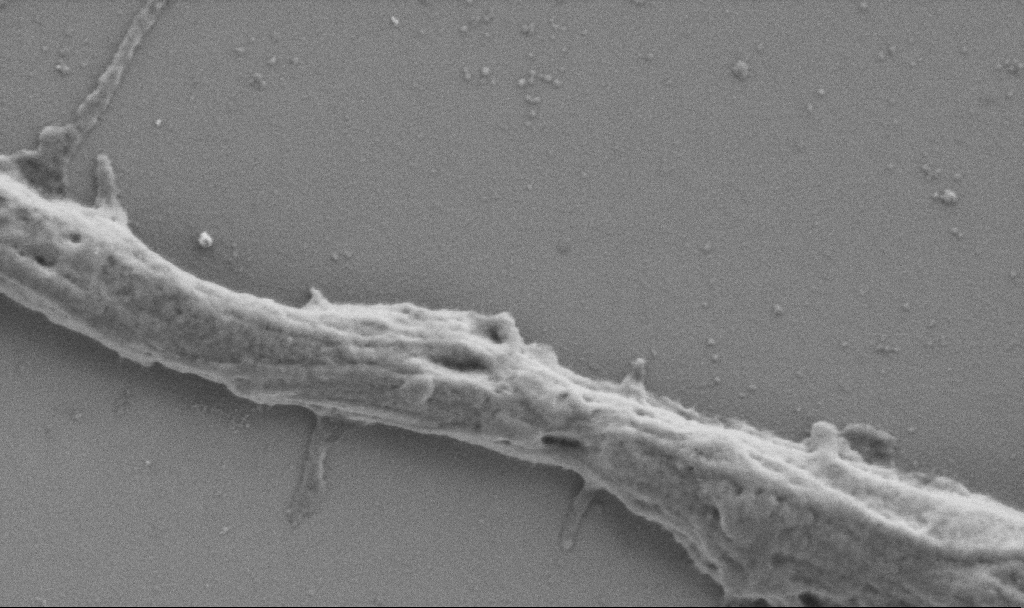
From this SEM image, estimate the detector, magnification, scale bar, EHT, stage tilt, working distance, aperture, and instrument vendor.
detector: SE2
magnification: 50 K X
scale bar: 1000 nm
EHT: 0.9 kV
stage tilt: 0°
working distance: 6.9 mm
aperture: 30 µm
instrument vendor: Zeiss